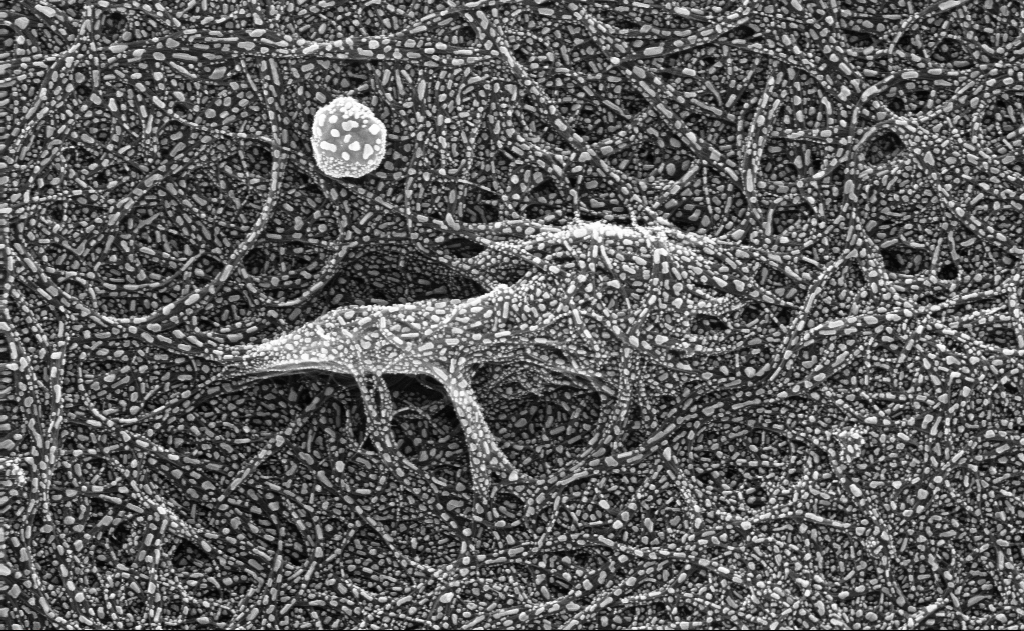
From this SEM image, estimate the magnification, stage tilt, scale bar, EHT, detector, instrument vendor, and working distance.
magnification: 104.26 K X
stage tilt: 0°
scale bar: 200 nm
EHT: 10 kV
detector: InLens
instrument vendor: Zeiss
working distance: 3 mm